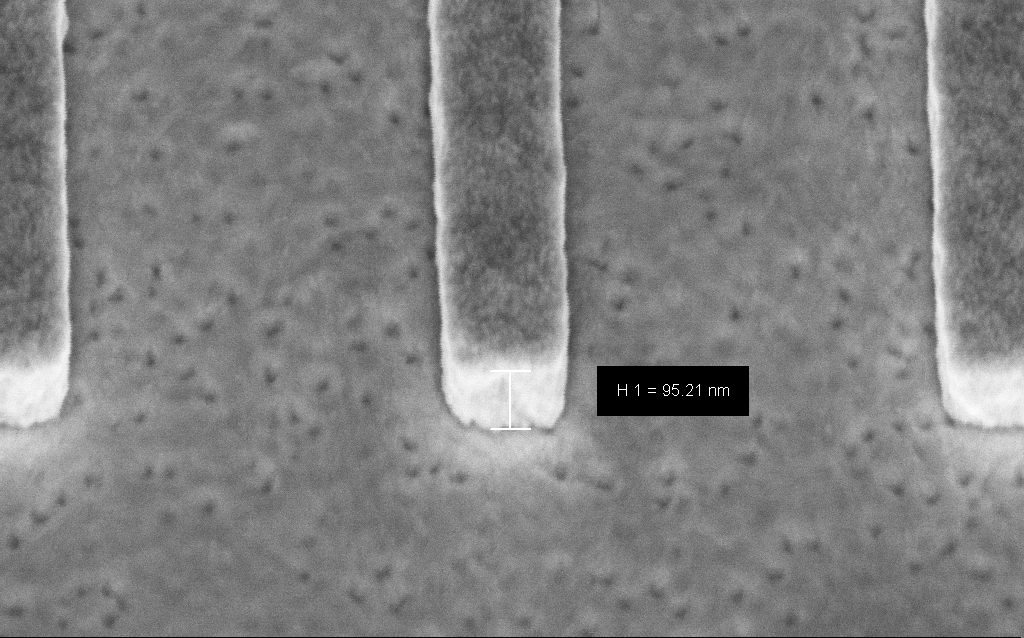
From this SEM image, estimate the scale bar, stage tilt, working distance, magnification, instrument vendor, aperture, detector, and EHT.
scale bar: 200 nm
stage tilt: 45°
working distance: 6.6 mm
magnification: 223.68 K X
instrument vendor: Zeiss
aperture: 30 µm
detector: InLens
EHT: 3 kV